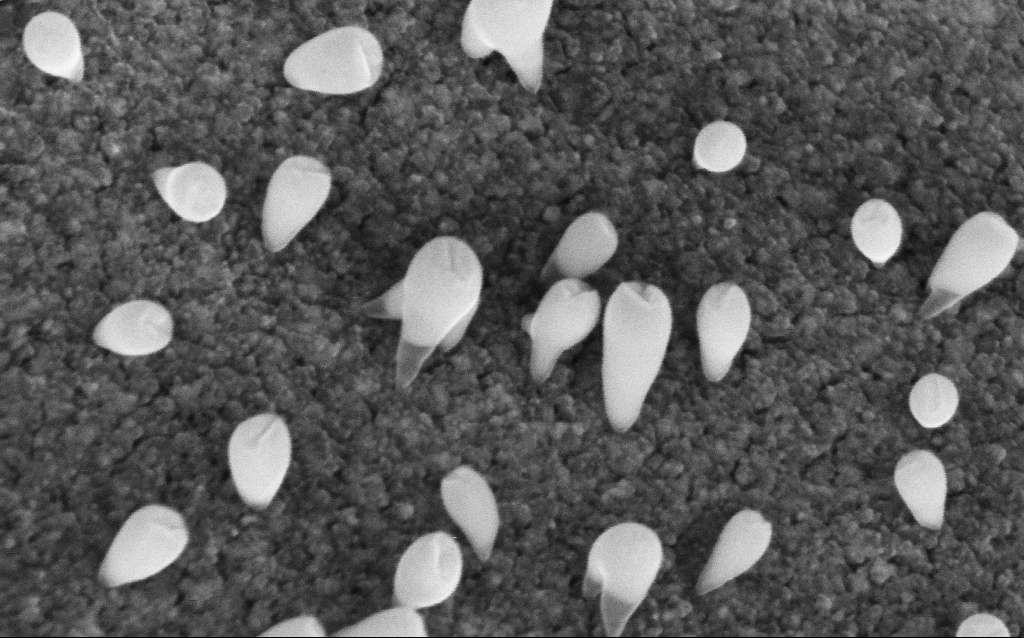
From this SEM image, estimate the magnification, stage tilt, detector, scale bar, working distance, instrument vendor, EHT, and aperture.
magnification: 236.74 K X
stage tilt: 42.7°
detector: InLens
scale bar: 200 nm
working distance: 6 mm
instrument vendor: Zeiss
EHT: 5 kV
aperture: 30 µm